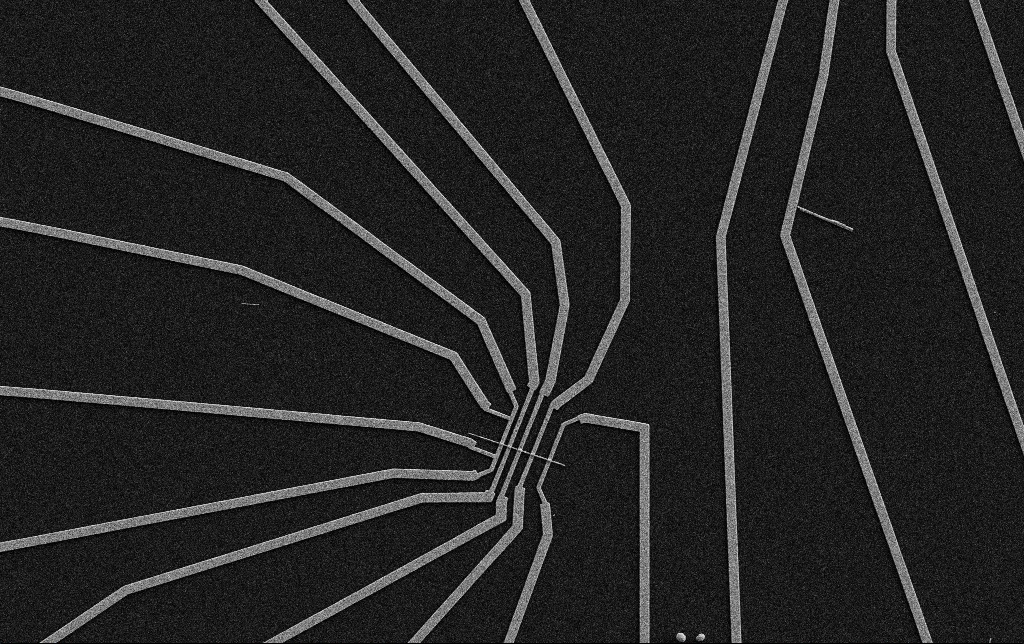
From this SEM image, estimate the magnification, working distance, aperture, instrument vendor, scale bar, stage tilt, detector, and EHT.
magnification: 5 K X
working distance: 10.7 mm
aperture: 30 µm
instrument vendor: Zeiss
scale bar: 10000 nm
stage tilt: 0°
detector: SE2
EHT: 5 kV